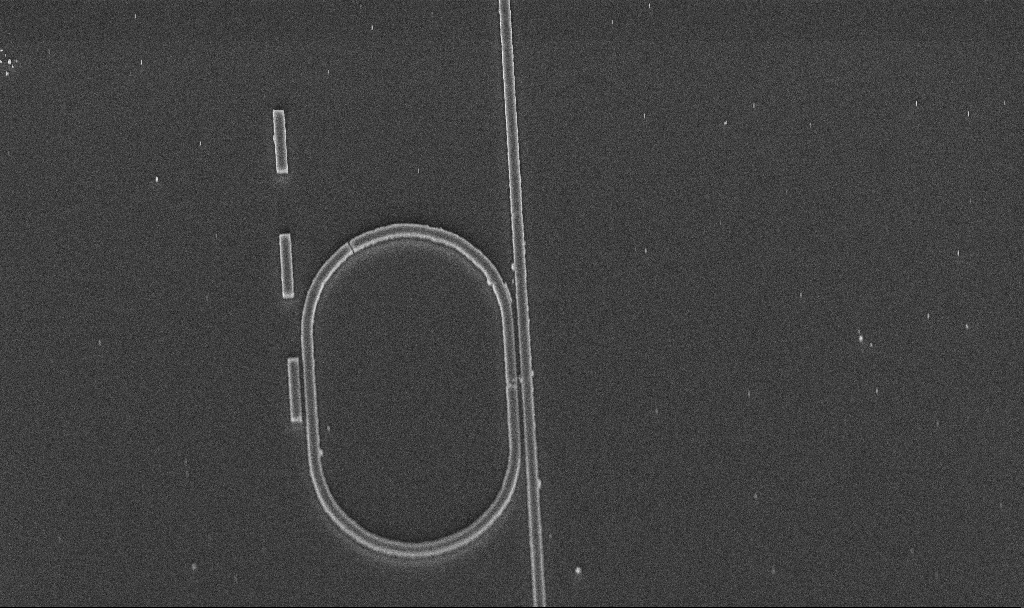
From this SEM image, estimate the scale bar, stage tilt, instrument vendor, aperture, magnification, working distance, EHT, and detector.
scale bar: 2000 nm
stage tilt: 45°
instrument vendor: Zeiss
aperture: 30 µm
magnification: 7.78 K X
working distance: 9.8 mm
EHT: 5 kV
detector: InLens